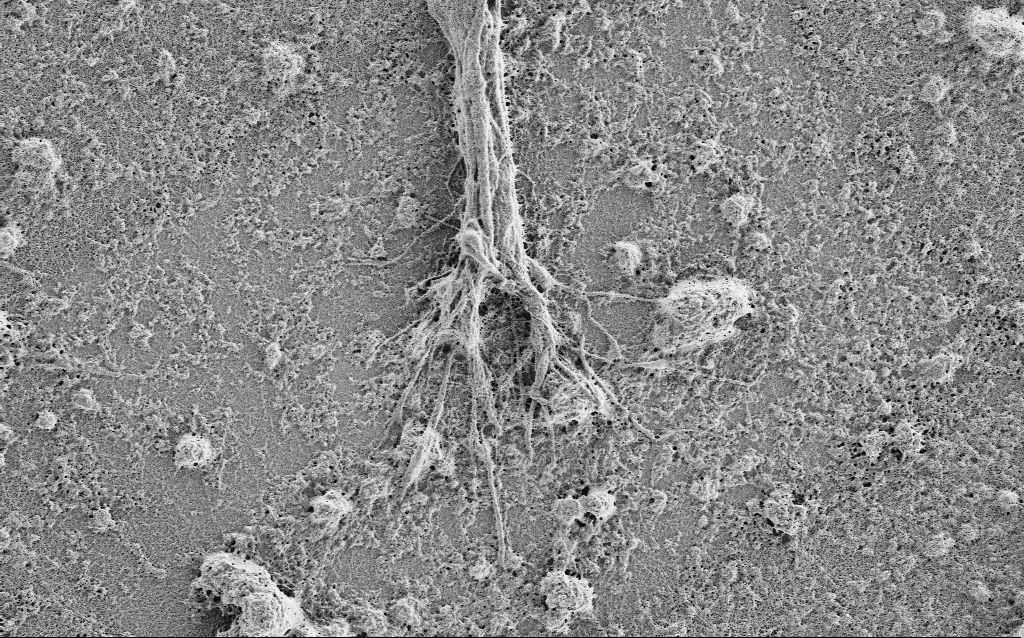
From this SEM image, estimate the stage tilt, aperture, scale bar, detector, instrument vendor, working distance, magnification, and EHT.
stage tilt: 0°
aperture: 30 µm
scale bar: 2000 nm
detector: SE2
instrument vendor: Zeiss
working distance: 4 mm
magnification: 7.5 K X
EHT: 1 kV